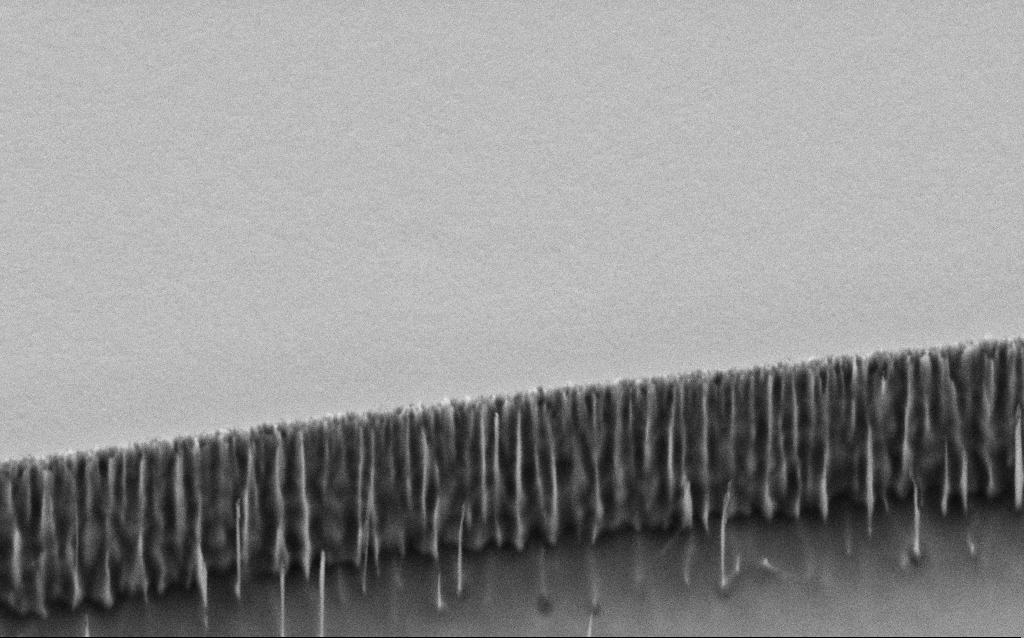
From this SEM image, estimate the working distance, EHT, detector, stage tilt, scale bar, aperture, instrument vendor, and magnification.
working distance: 6 mm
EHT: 3 kV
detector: SE2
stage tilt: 45°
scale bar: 1000 nm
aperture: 30 µm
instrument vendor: Zeiss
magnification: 48.23 K X